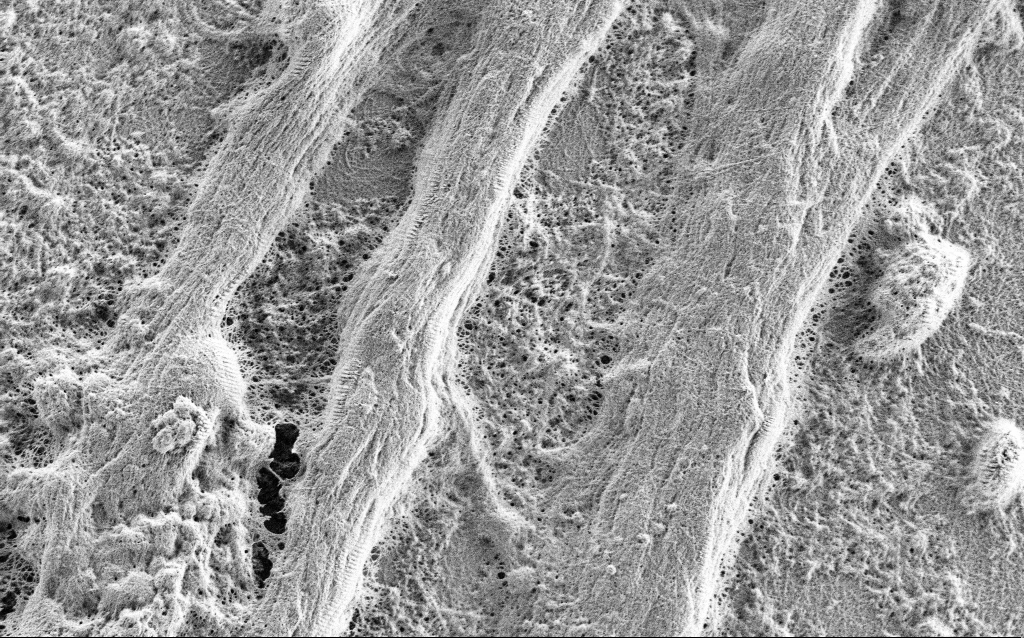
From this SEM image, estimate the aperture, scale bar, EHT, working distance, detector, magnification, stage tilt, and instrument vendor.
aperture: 30 µm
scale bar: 2000 nm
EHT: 0.9 kV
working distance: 4 mm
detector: SE2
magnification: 10 K X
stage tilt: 0°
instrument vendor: Zeiss